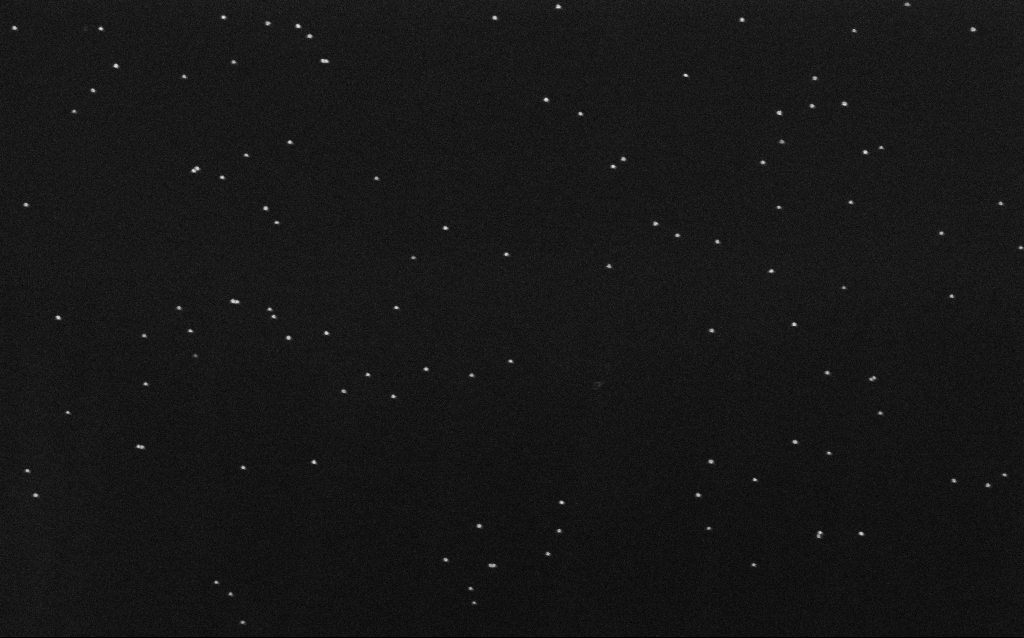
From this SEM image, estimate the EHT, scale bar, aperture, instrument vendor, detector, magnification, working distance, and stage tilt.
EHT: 10 kV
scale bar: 200 nm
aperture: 30 µm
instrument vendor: Zeiss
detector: InLens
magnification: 100 K X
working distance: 6.5 mm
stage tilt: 0°